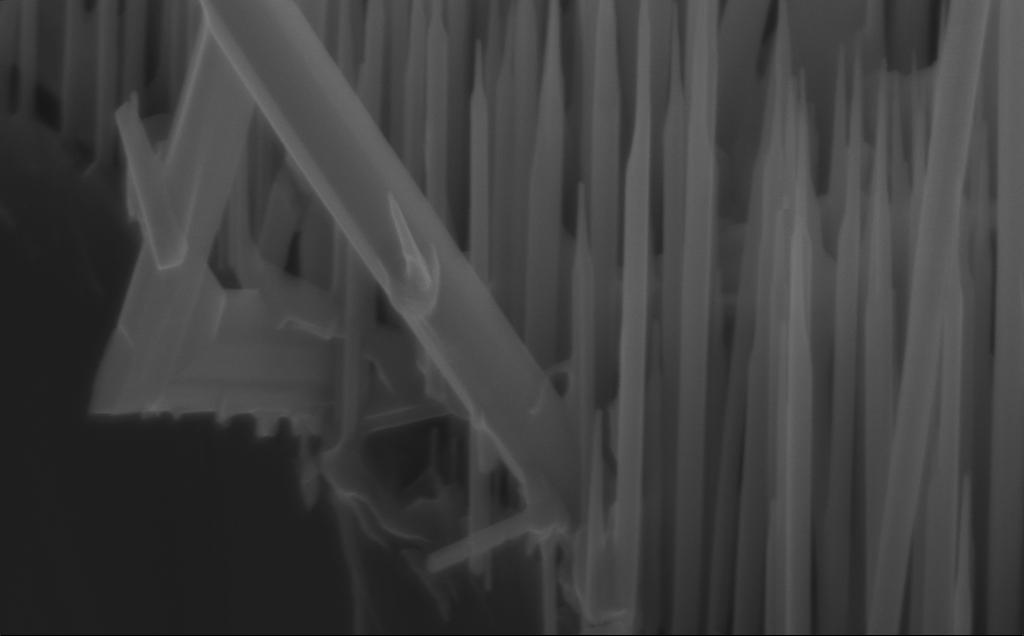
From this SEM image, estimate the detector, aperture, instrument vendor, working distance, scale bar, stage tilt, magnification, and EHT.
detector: InLens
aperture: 30 µm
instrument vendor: Zeiss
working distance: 5 mm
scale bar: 200 nm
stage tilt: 45°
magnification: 108.81 K X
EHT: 10 kV